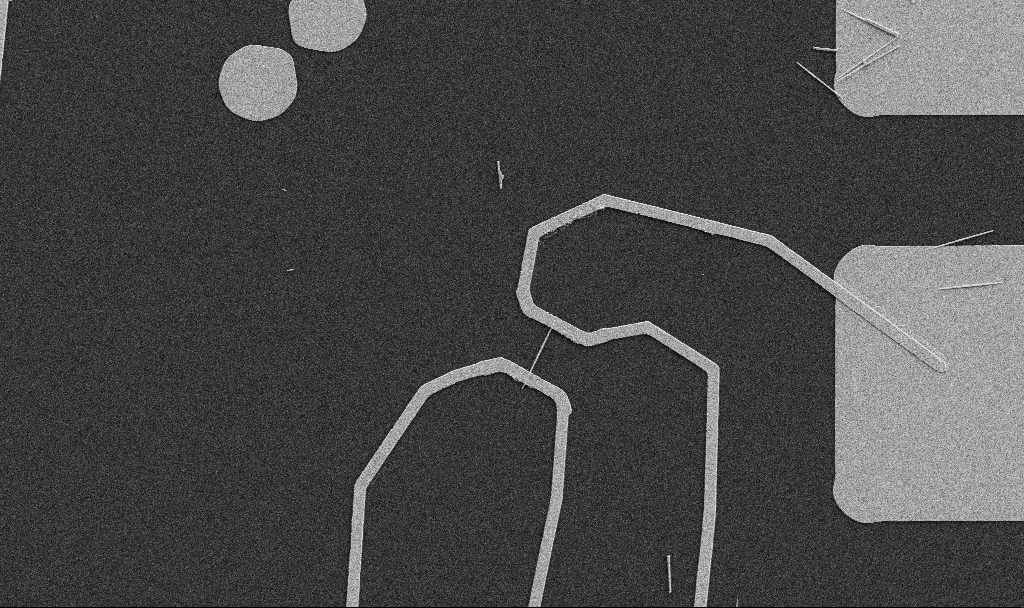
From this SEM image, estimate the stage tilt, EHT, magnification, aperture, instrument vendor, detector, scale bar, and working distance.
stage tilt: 0°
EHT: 5 kV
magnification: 5 K X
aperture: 30 µm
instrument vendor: Zeiss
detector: SE2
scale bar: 10000 nm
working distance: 10.7 mm